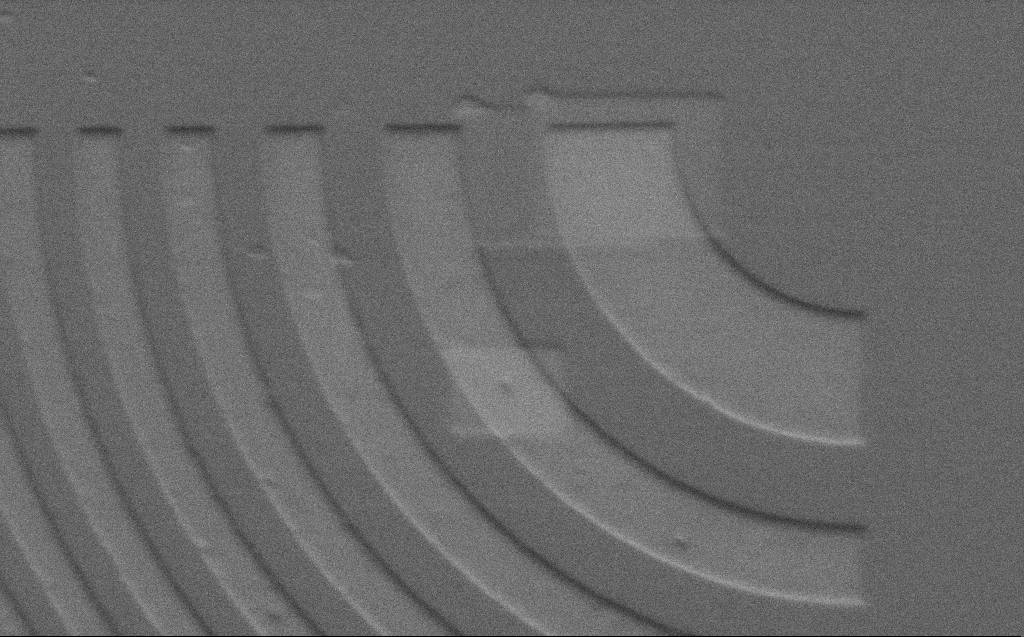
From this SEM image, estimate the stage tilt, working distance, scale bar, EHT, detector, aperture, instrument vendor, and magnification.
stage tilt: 45°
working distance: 4 mm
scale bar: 1000 nm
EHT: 2 kV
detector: SE2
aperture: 30 µm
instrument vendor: Zeiss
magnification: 12.35 K X